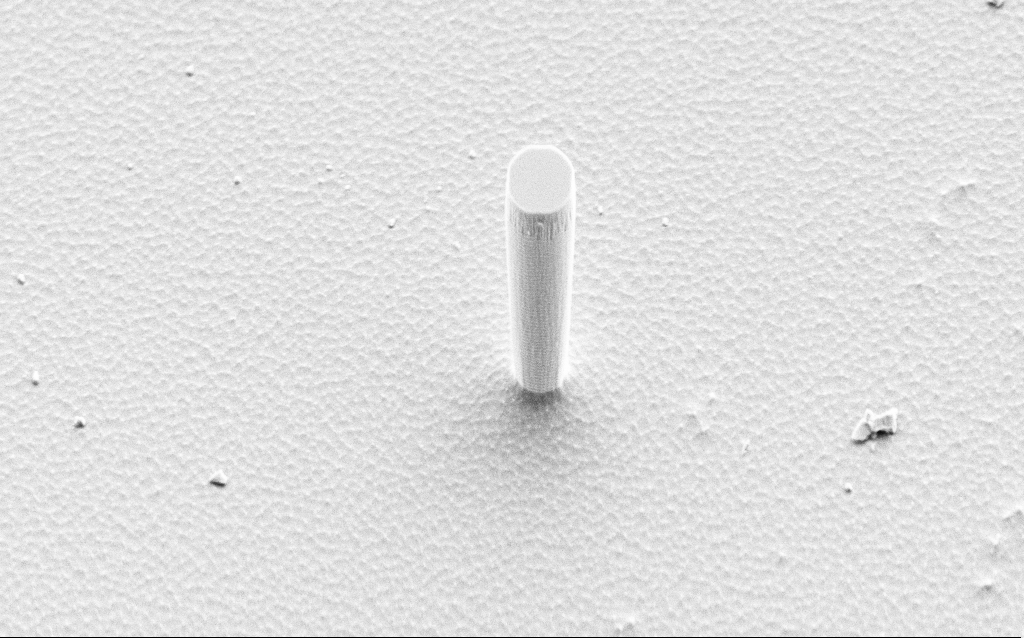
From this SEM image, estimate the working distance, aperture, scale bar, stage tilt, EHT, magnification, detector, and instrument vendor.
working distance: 10 mm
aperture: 30 µm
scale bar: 10000 nm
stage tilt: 50°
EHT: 5 kV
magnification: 3.8 K X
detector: SE2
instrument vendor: Zeiss